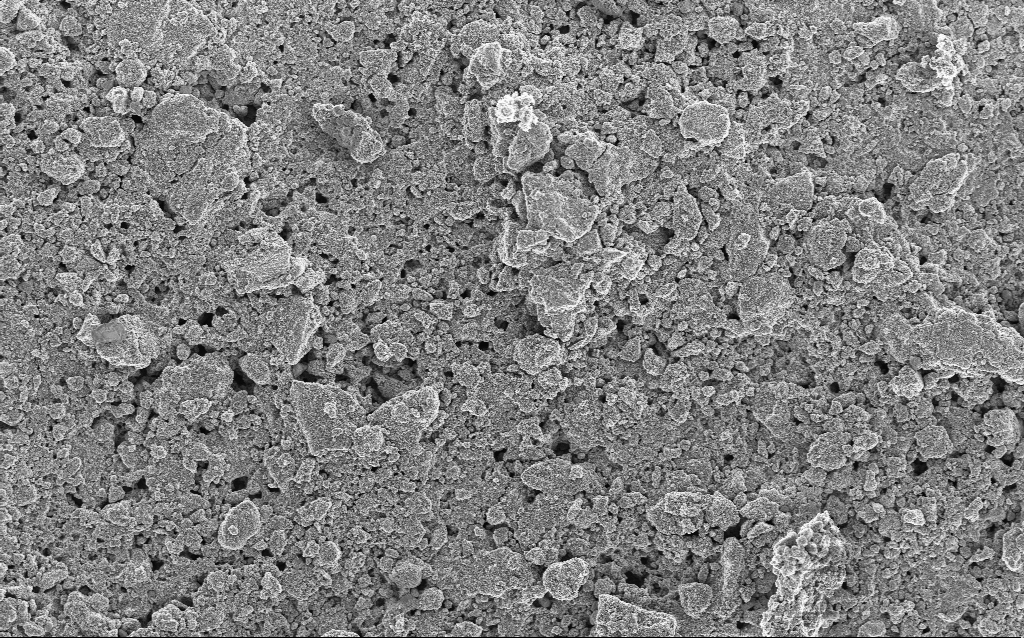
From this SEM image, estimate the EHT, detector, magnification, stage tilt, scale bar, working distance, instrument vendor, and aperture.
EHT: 5 kV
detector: InLens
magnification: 1.23 K X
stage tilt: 0°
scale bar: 10000 nm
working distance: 4.5 mm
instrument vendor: Zeiss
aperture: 30 µm